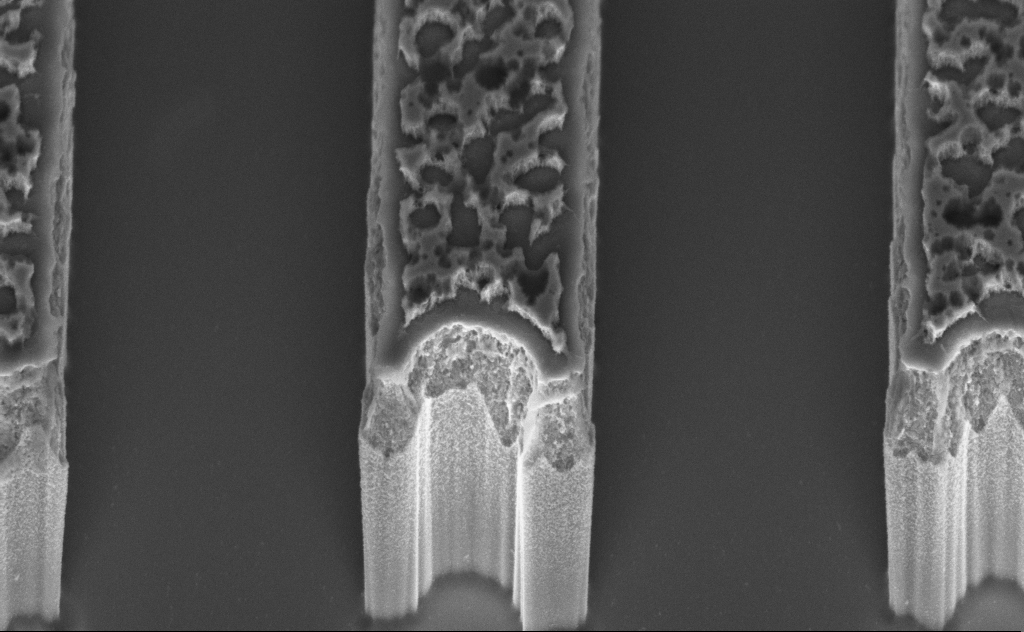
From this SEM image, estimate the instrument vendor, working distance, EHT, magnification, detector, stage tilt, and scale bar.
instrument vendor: Zeiss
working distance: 8 mm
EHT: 3 kV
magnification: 48.17 K X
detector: InLens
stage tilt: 45°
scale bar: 1000 nm